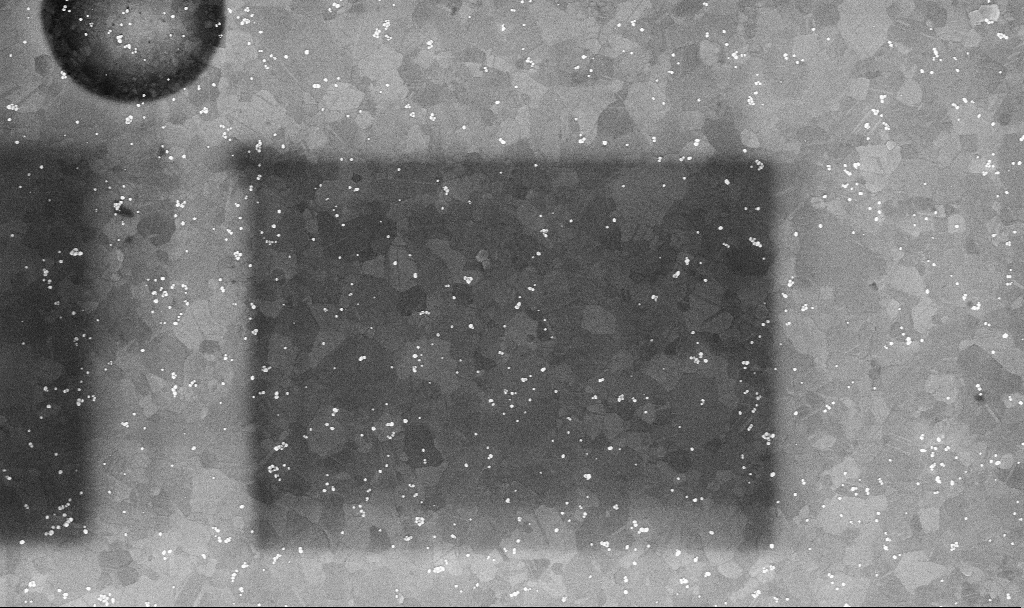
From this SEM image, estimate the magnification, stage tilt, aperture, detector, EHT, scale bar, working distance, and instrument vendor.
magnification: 50.84 K X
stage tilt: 0°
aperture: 30 µm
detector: InLens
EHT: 10 kV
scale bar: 1000 nm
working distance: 3.4 mm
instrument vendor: Zeiss